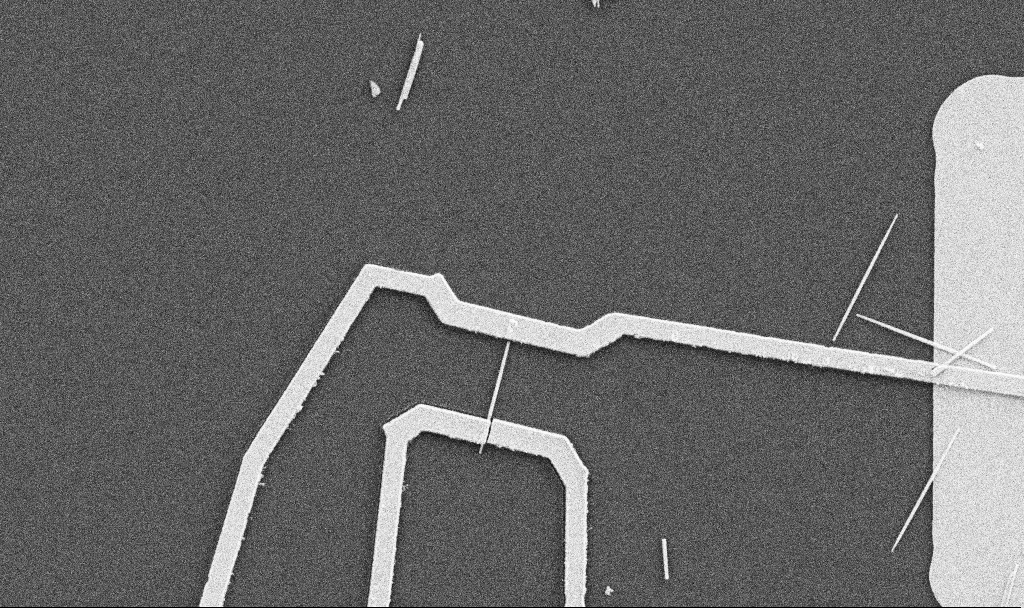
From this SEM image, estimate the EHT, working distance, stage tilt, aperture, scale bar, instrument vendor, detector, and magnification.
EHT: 5 kV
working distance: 10.7 mm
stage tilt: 0°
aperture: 30 µm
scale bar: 2000 nm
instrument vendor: Zeiss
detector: SE2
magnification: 10 K X